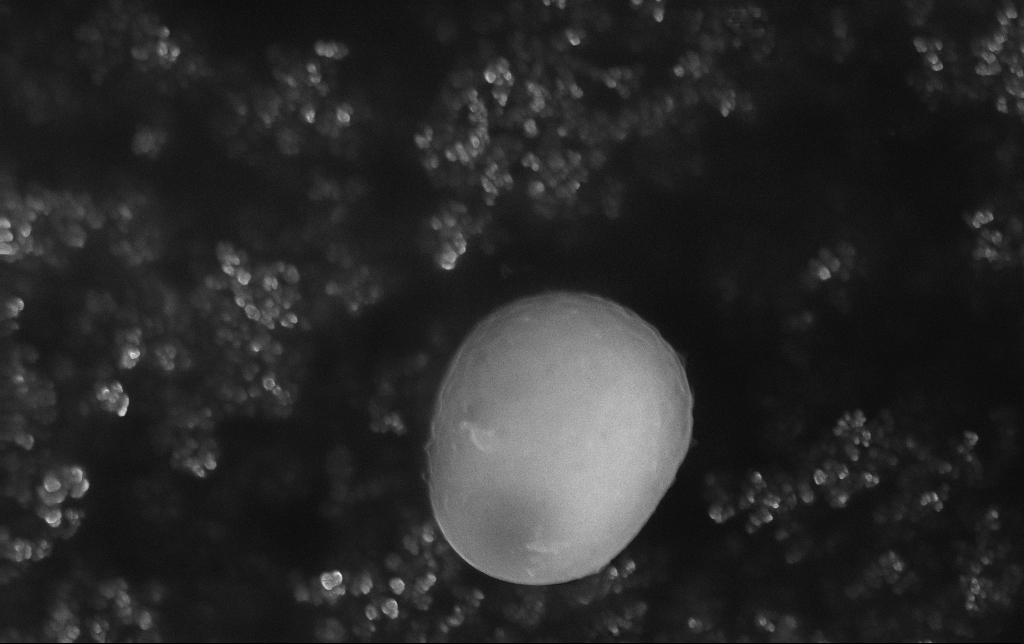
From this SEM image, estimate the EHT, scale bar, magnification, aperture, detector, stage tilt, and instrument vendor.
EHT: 15 kV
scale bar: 1000 nm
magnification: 39.81 K X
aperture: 30 µm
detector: InLens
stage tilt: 0°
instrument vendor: Zeiss